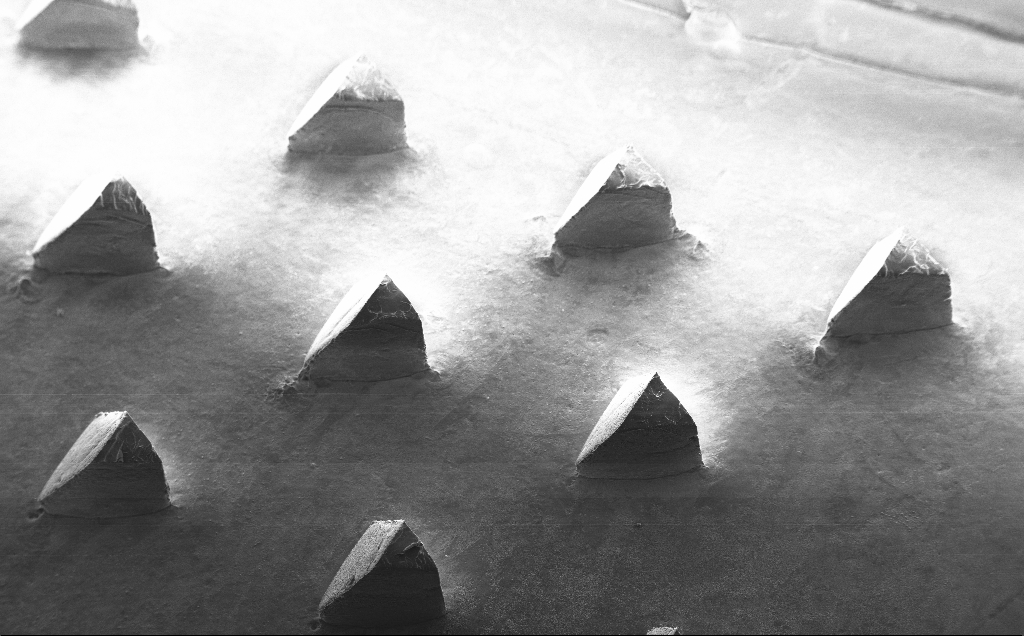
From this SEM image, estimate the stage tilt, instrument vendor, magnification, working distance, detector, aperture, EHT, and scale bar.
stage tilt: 30°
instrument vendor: Zeiss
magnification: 0.08 K X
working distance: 9 mm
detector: SE2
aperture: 30 µm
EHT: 10 kV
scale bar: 200000 nm